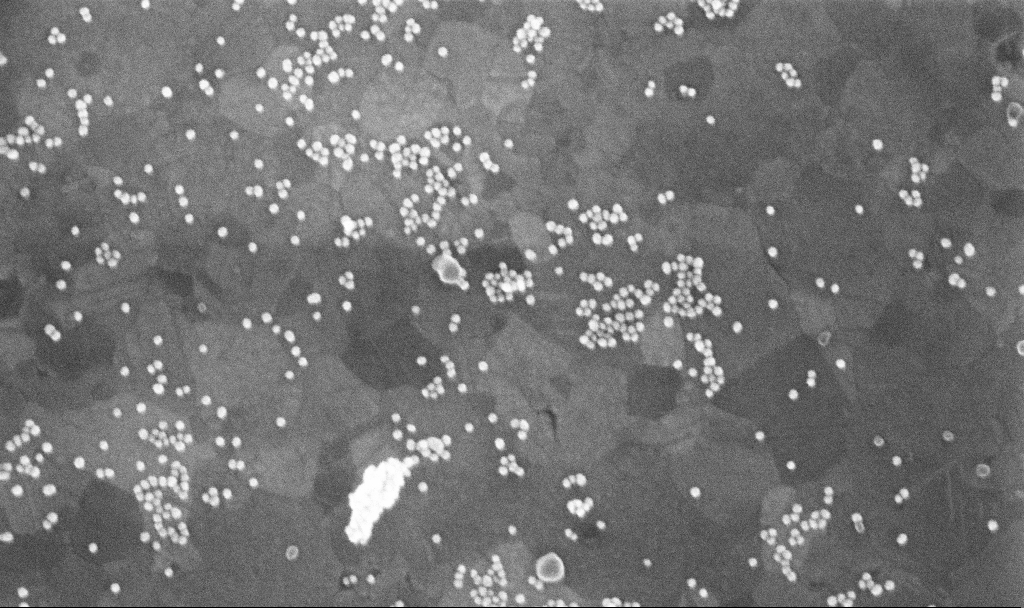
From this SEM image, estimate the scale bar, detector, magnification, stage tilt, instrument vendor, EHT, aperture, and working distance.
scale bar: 200 nm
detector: InLens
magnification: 177.15 K X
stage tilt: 0°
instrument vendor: Zeiss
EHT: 10 kV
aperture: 30 µm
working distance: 3.7 mm